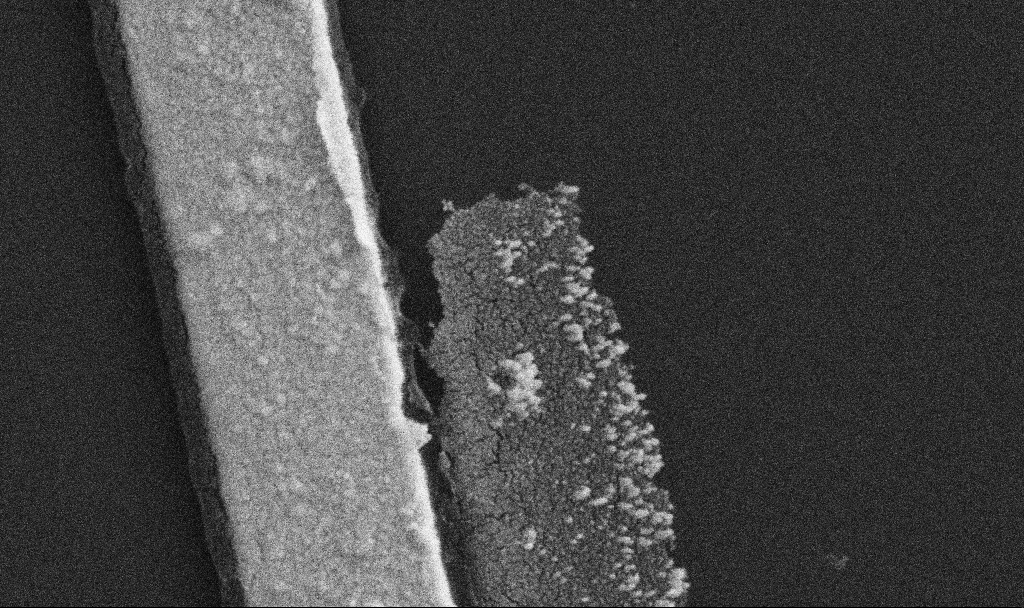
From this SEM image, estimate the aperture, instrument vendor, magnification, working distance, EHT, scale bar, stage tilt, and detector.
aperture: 30 µm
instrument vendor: Zeiss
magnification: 105.2 K X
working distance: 10.7 mm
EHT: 5 kV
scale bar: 200 nm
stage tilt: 0°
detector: SE2